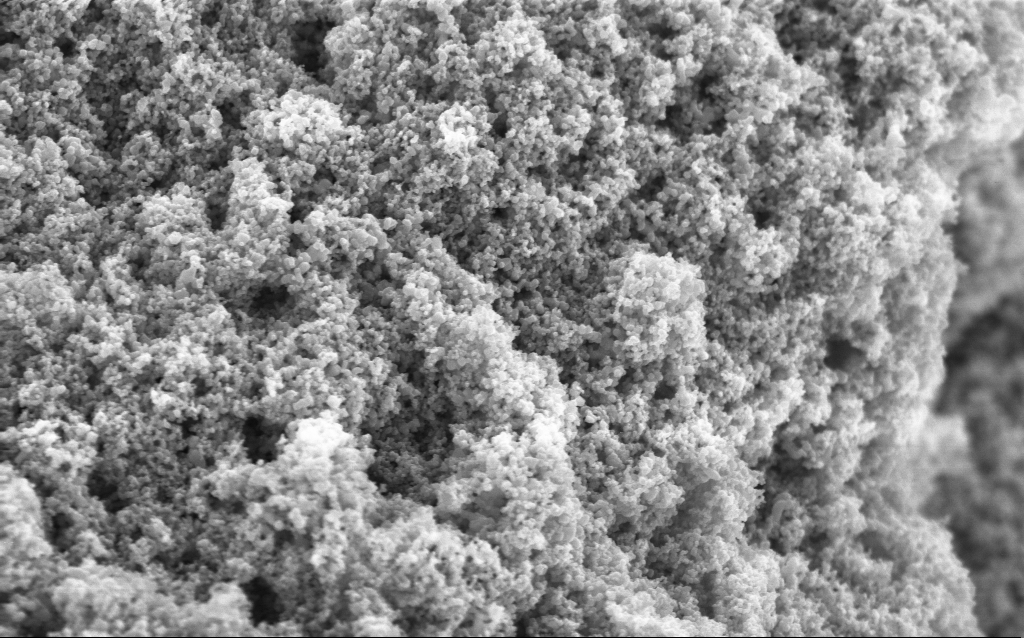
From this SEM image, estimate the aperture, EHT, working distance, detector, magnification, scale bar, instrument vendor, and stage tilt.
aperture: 30 µm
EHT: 5 kV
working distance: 4.5 mm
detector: InLens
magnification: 68.65 K X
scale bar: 1000 nm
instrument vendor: Zeiss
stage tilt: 0°